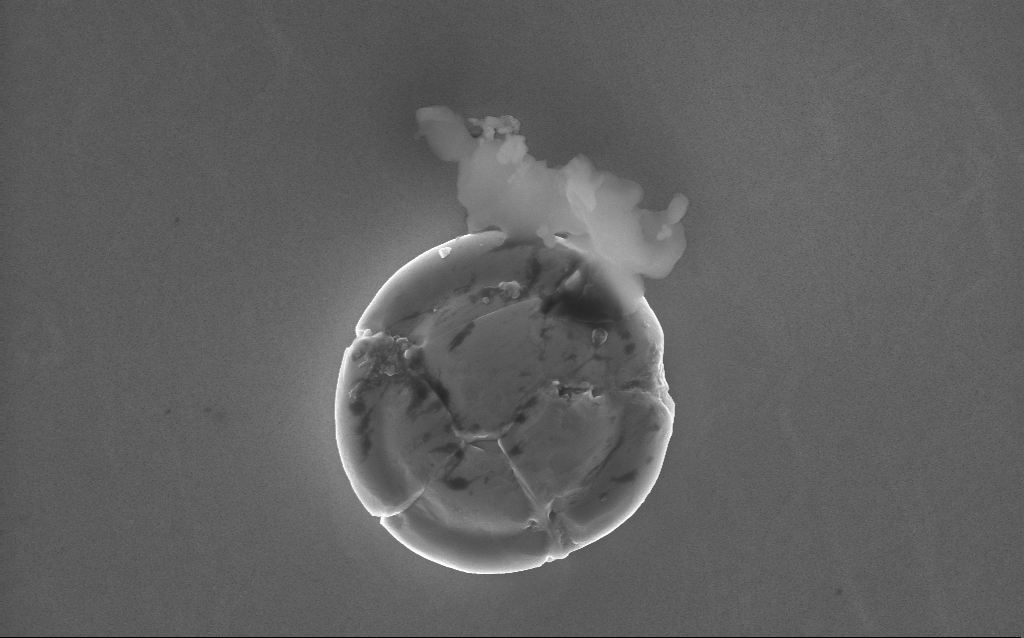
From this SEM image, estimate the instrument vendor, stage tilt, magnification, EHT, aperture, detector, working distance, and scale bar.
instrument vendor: Zeiss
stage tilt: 0°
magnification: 30.82 K X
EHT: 10 kV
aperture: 30 µm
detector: InLens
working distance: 2 mm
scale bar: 2000 nm